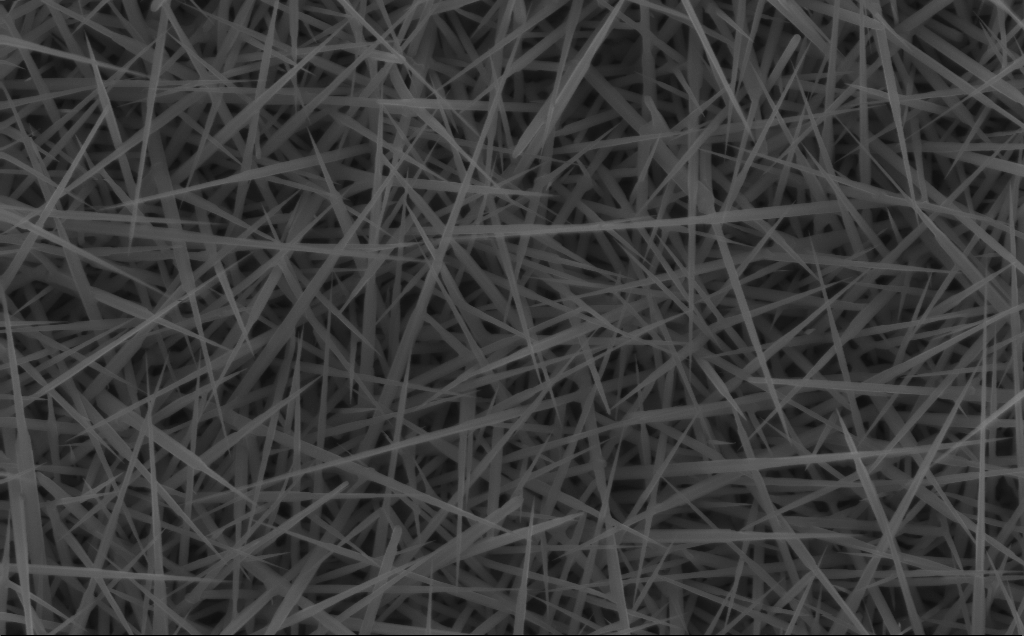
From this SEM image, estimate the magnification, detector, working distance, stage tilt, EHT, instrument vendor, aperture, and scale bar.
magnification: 40 K X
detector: InLens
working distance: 4 mm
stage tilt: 0°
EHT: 10 kV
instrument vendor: Zeiss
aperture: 30 µm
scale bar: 1000 nm